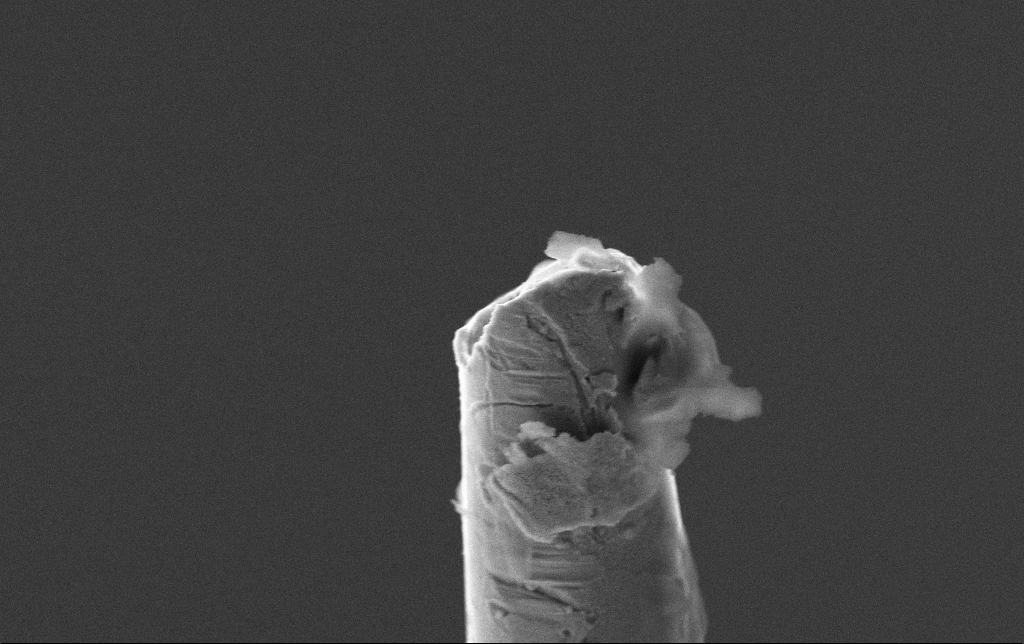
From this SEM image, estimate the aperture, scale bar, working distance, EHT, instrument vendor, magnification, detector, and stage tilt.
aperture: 30 µm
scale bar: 1000 nm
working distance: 5.6 mm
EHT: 10 kV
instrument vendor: Zeiss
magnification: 15.36 K X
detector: SE2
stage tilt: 27.9°